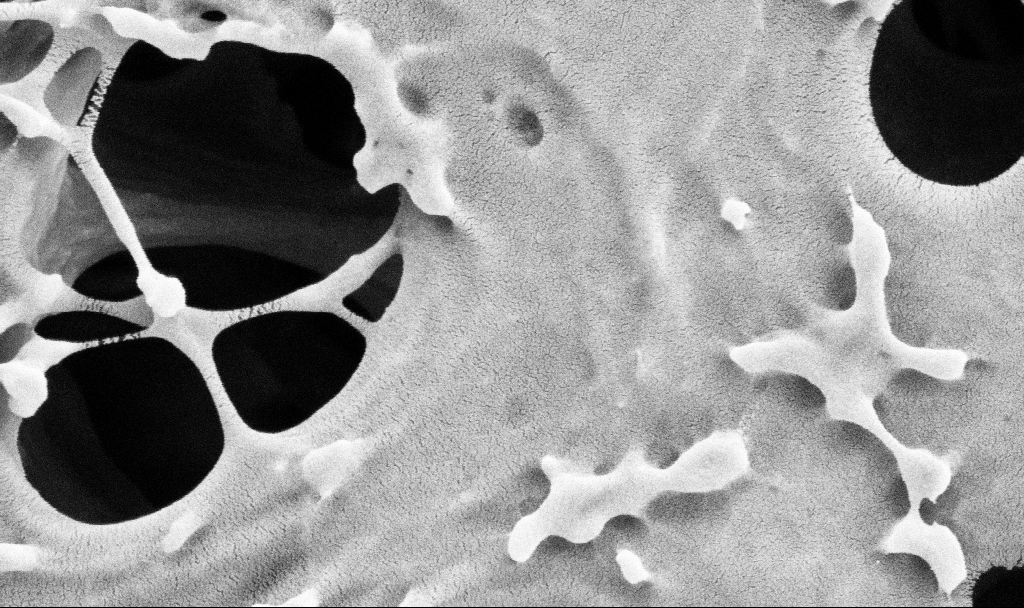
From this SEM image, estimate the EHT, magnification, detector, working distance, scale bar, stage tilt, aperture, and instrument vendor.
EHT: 2 kV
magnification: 100 K X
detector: SE2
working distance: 3.7 mm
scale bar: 200 nm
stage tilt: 0°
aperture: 30 µm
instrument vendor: Zeiss